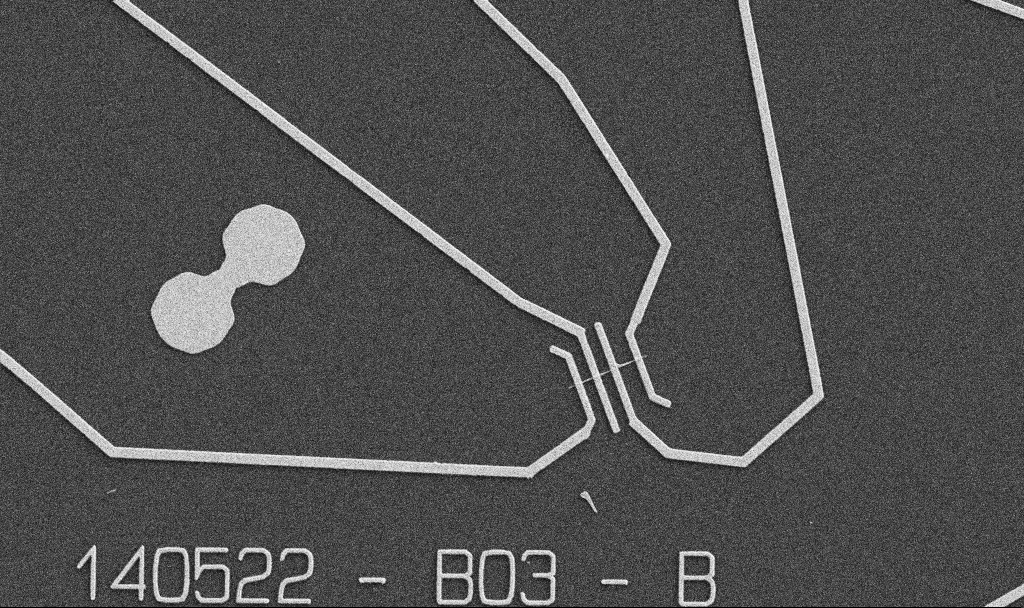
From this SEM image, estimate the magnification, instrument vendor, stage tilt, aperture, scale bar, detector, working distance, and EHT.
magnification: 5 K X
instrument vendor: Zeiss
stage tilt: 0°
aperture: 30 µm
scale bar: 10000 nm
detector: SE2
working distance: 10.7 mm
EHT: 5 kV